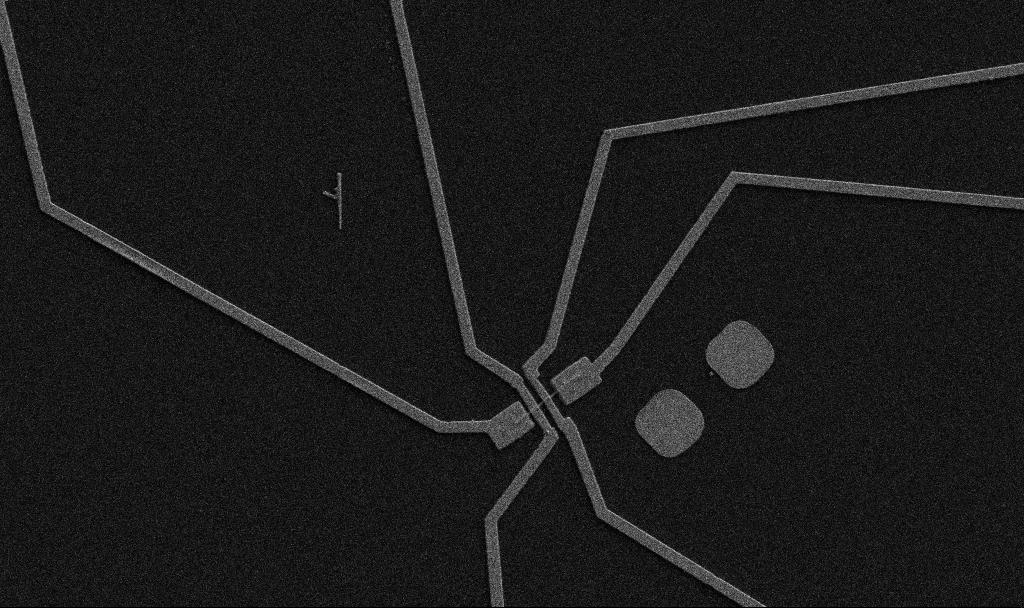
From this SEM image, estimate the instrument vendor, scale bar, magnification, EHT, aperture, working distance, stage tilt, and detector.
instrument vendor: Zeiss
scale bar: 10000 nm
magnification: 5 K X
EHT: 5 kV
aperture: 30 µm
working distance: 10.7 mm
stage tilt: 0°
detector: SE2